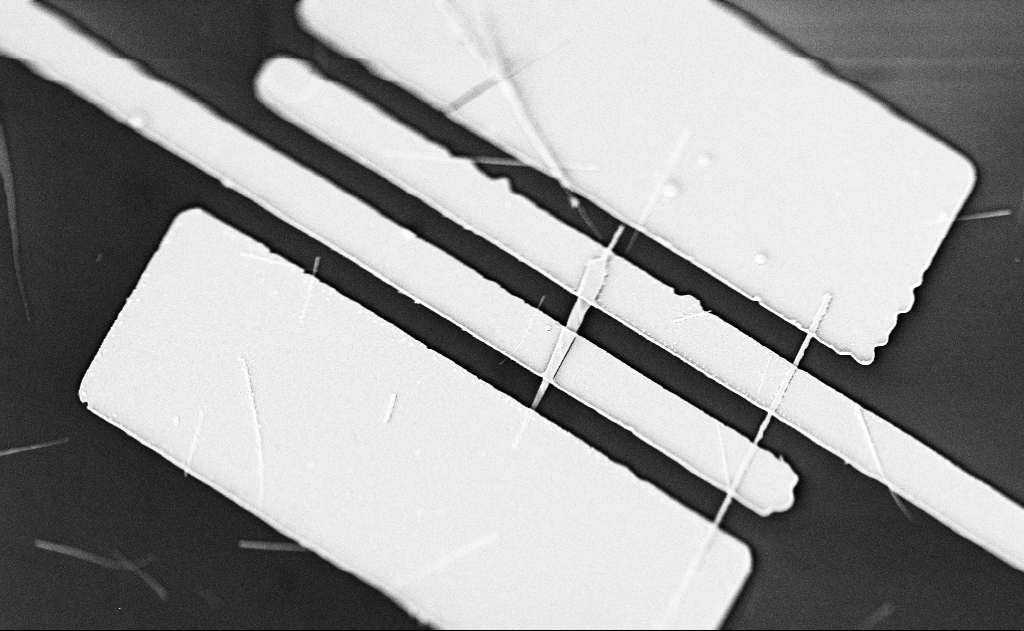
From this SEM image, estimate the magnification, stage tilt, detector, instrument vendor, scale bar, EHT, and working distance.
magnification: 8 K X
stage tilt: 0°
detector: SE2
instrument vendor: Zeiss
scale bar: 2000 nm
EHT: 5 kV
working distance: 20 mm